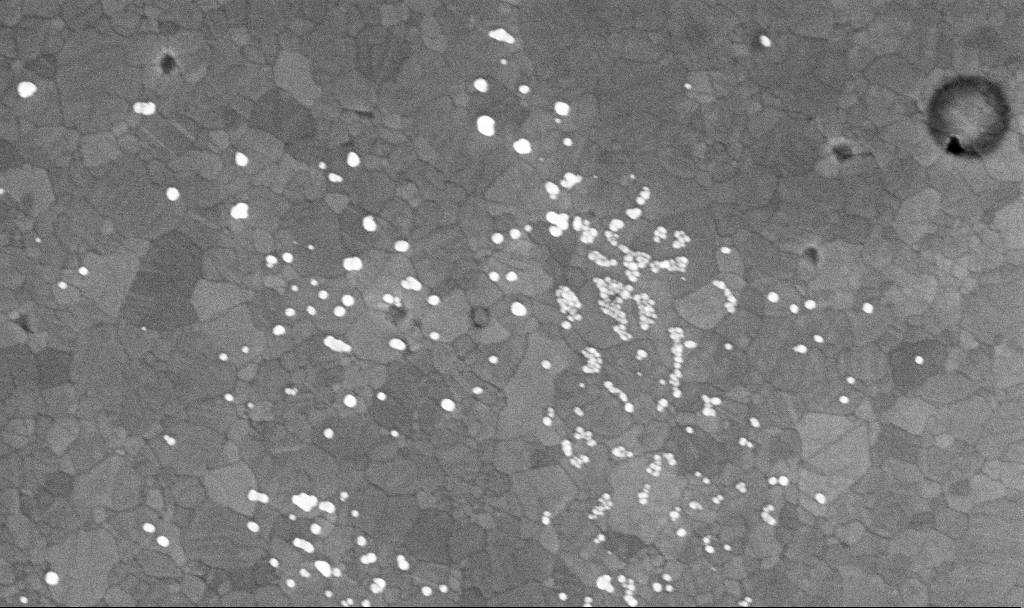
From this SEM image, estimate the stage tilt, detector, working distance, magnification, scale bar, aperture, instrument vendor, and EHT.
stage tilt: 0°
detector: InLens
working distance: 3.4 mm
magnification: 78.04 K X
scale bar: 200 nm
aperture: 30 µm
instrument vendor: Zeiss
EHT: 10 kV